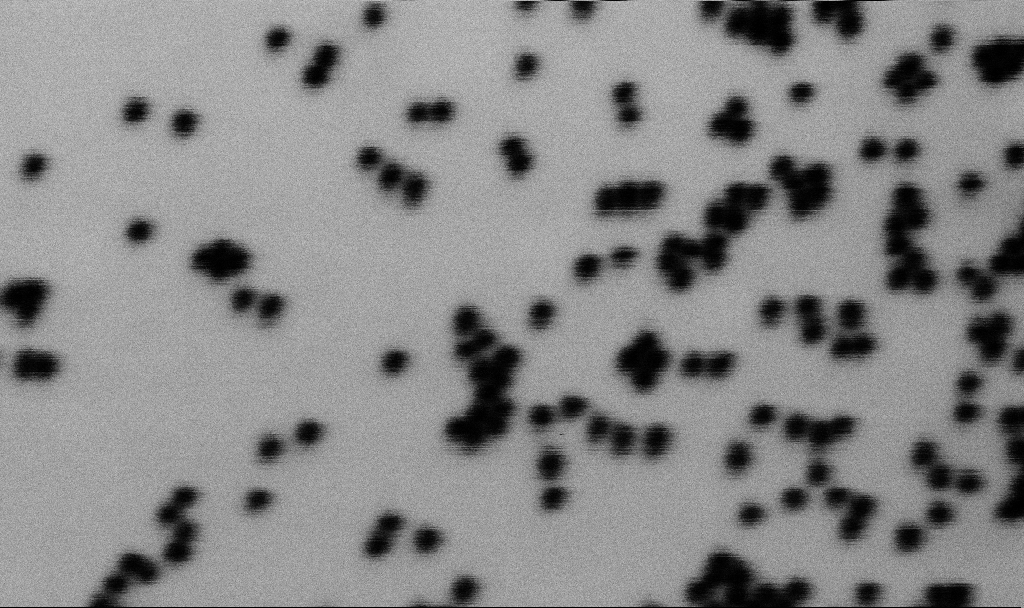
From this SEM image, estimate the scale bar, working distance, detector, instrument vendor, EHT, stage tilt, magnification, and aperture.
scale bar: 100 nm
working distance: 6.5 mm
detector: SE2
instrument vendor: Zeiss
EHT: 2 kV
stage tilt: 0°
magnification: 374 K X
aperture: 30 µm